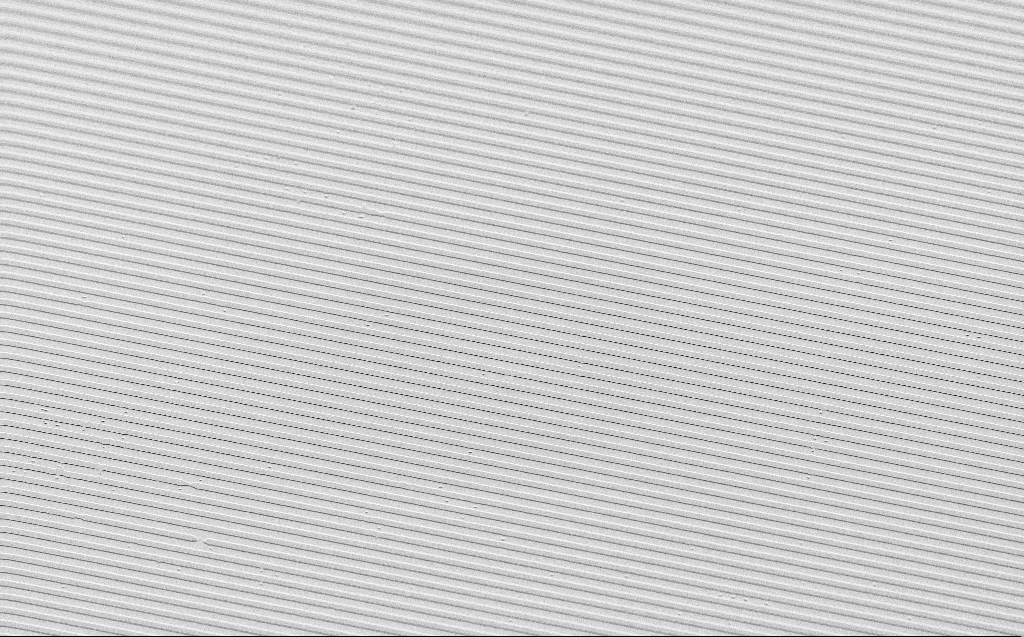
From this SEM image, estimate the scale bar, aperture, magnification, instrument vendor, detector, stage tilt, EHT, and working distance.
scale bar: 10000 nm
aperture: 30 µm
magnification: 1.22 K X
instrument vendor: Zeiss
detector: SE2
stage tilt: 45°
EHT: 3 kV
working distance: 9 mm